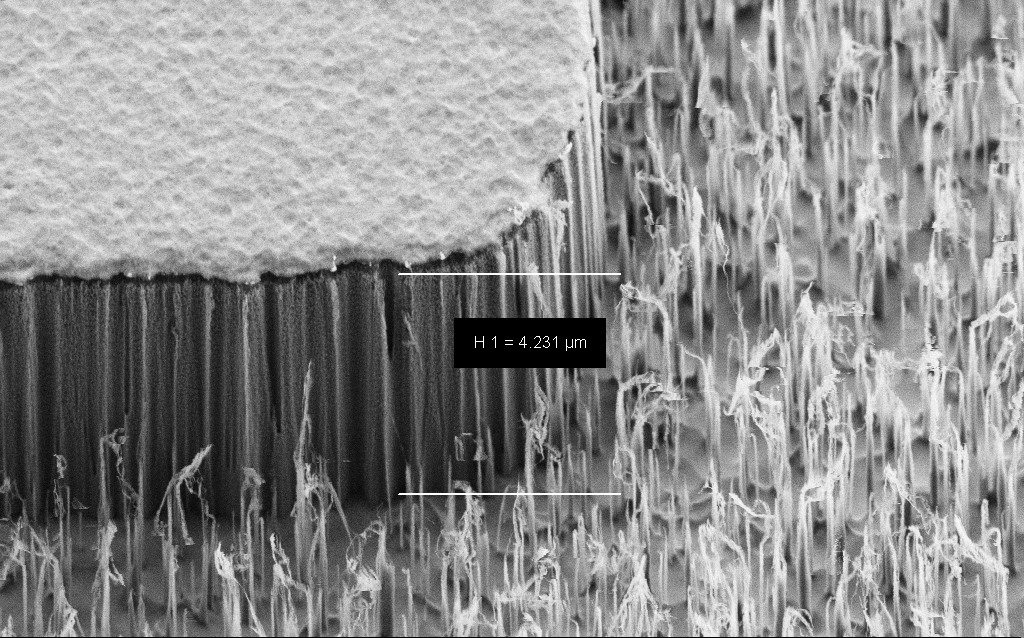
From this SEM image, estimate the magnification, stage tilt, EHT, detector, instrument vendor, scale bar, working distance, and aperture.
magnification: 19.09 K X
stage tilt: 45°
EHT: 2 kV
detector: SE2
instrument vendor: Zeiss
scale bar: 2000 nm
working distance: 7 mm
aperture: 30 µm